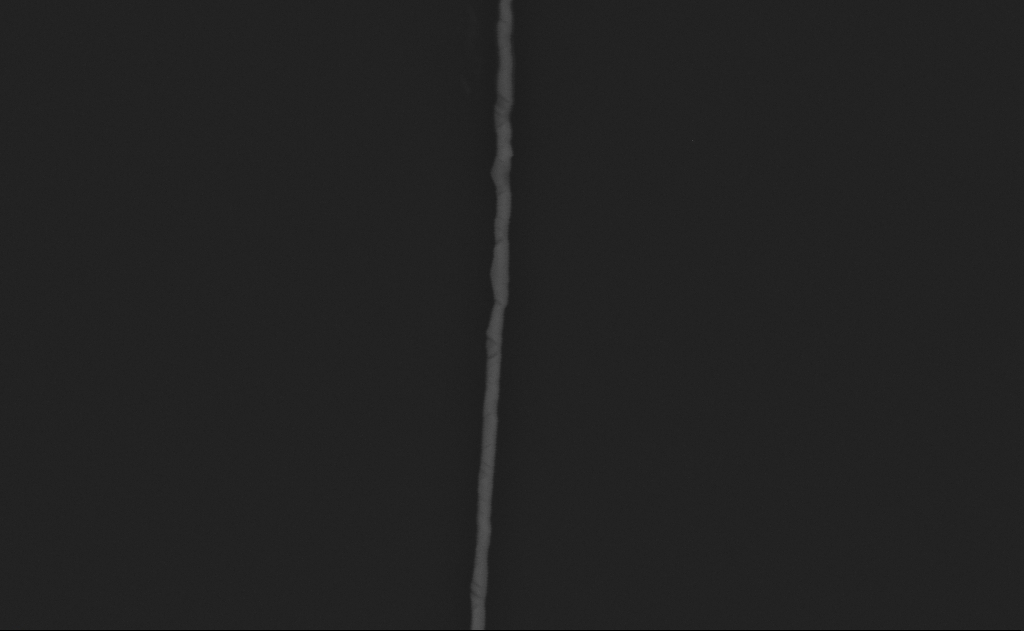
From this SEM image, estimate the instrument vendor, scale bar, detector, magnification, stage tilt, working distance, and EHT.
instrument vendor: Zeiss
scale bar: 1000 nm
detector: SE2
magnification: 60.93 K X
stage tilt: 0°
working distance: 12 mm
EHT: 5 kV